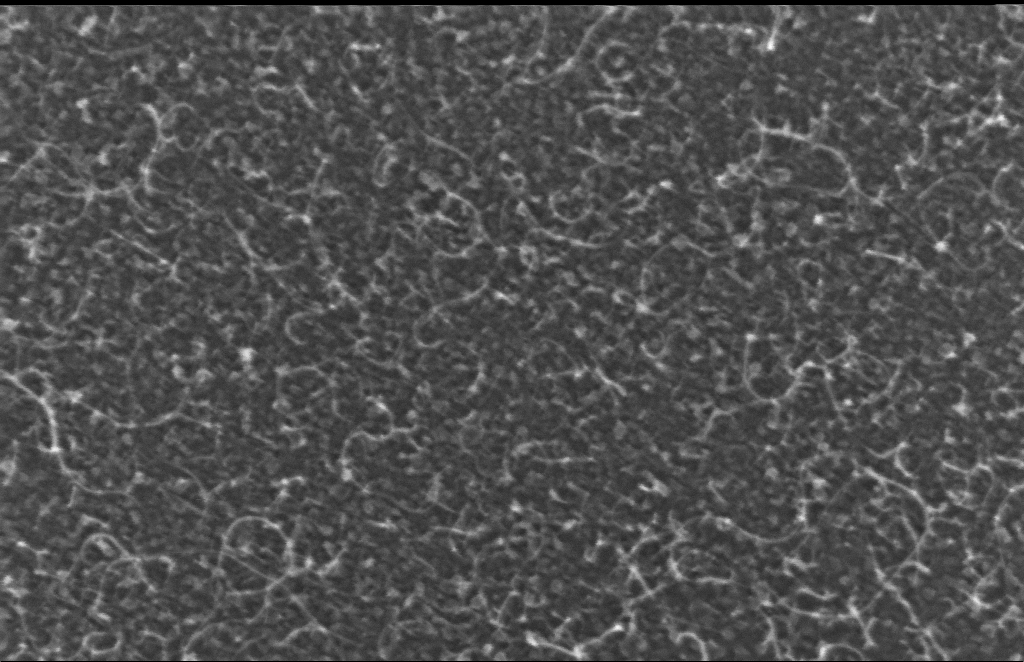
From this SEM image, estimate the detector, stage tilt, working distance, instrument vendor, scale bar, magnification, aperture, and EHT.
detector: InLens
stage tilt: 0°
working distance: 6 mm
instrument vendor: Zeiss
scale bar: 100 nm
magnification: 329.7 K X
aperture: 30 µm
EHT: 5 kV